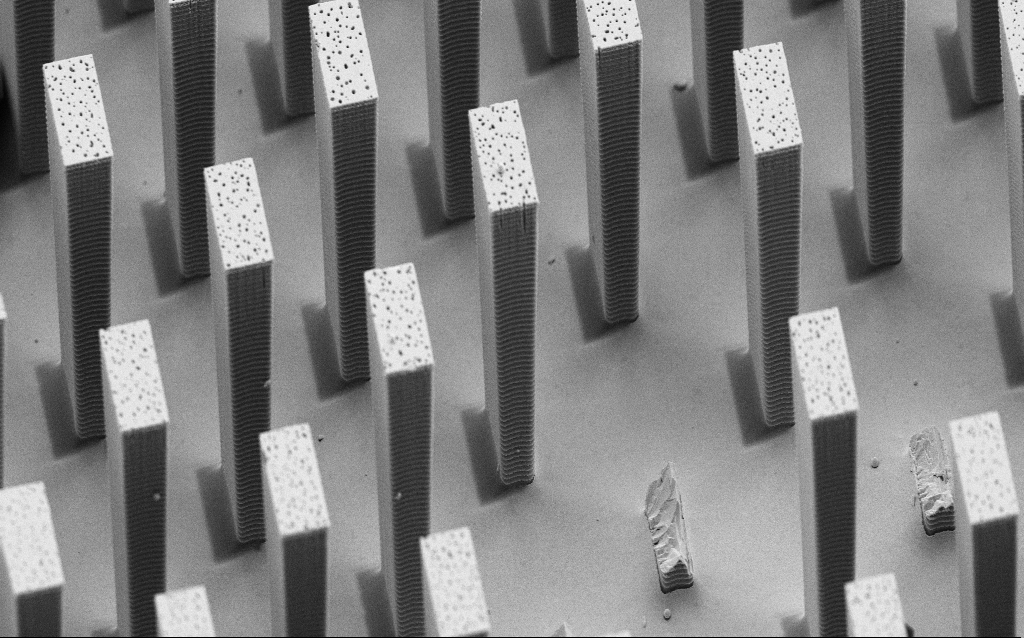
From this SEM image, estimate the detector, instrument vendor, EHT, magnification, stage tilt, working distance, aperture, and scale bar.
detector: SE2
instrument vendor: Zeiss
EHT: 5 kV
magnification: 4.94 K X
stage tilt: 45°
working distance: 8 mm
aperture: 30 µm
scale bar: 10000 nm